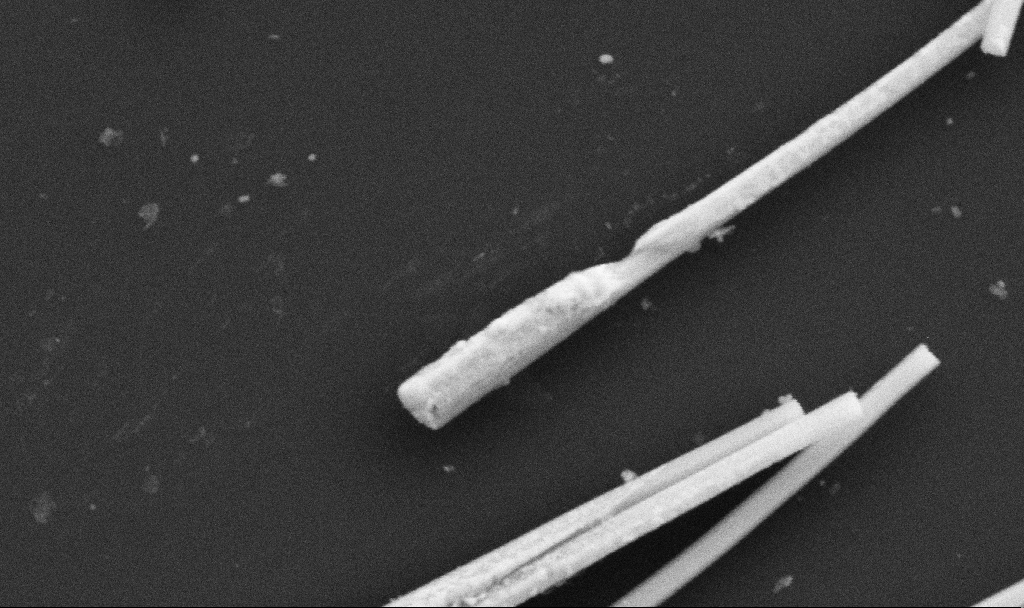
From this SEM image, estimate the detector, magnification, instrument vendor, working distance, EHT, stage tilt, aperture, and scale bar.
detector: SE2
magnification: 105.2 K X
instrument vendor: Zeiss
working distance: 10.7 mm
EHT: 5 kV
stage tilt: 0°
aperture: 30 µm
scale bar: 200 nm